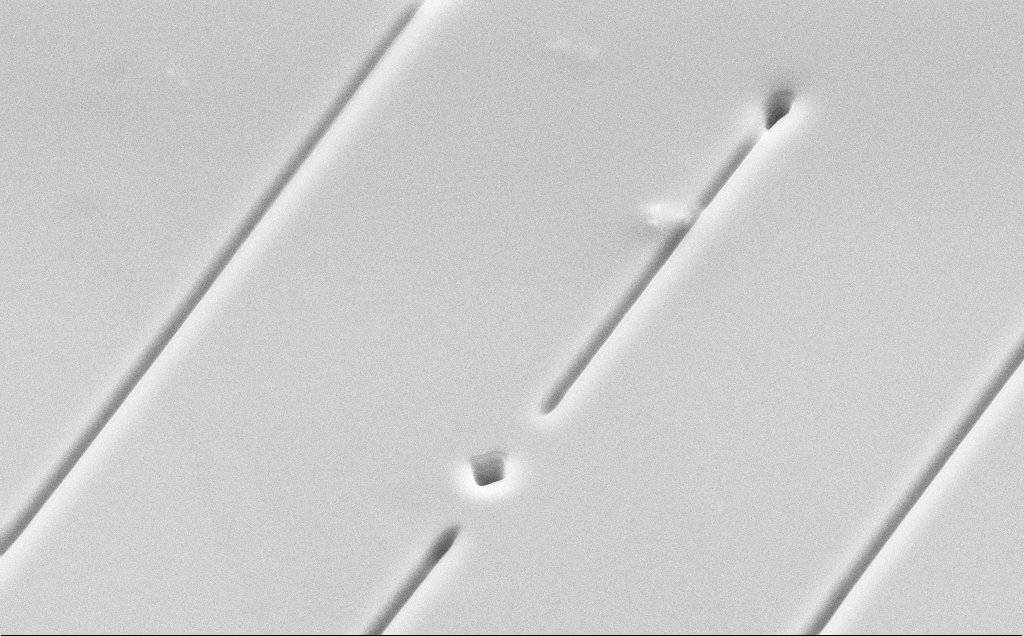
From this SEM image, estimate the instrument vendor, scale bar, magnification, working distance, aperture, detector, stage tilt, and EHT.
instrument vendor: Zeiss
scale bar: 10000 nm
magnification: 6.99 K X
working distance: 13 mm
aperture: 30 µm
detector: SE2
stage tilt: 45°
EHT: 10 kV